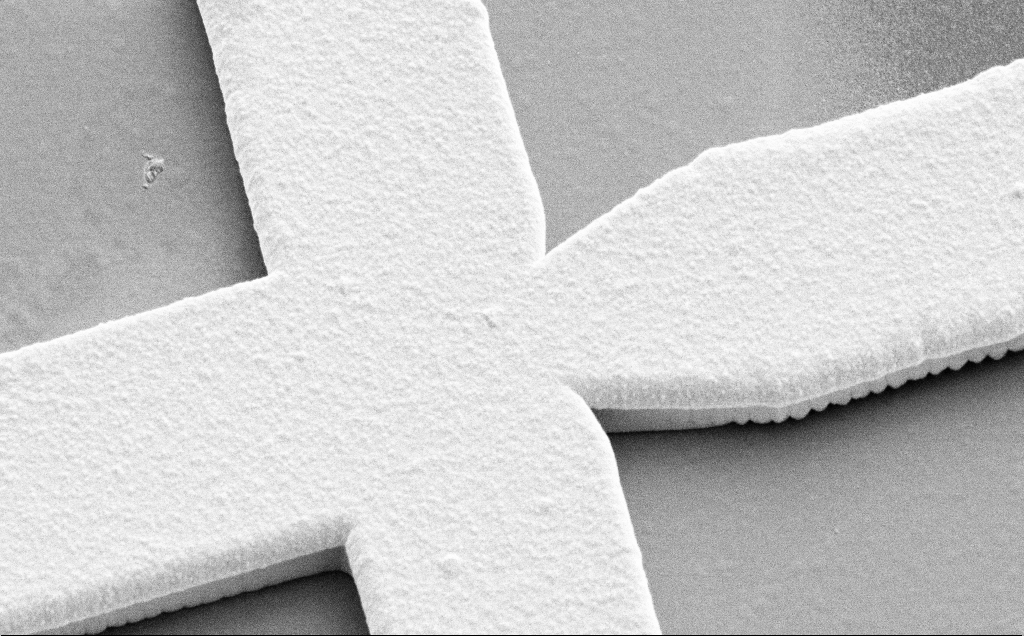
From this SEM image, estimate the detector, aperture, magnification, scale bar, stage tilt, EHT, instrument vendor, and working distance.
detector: SE2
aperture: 30 µm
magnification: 8.78 K X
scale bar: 2000 nm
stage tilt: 45°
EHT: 10 kV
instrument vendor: Zeiss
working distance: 12 mm